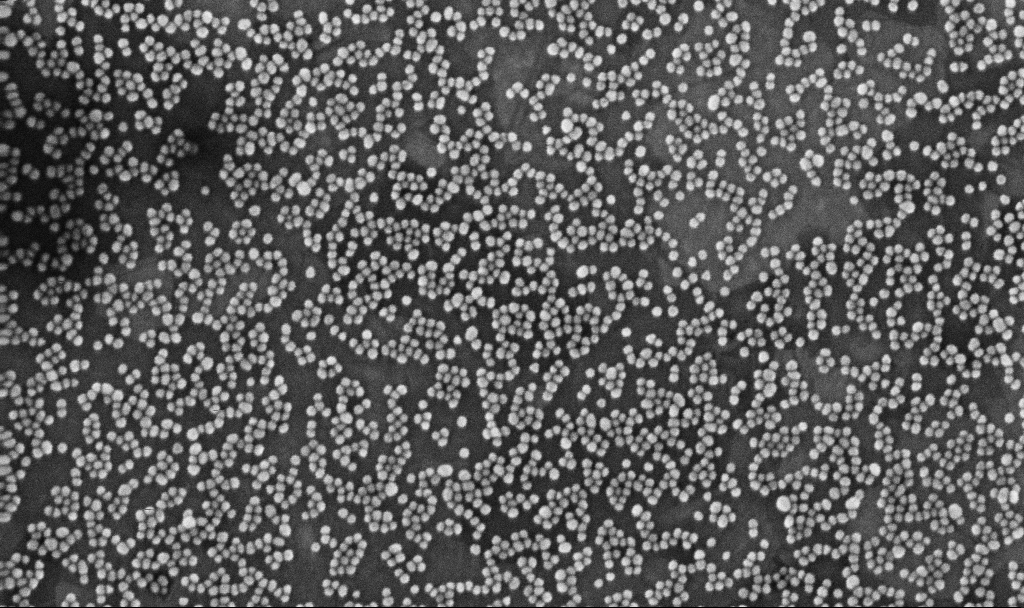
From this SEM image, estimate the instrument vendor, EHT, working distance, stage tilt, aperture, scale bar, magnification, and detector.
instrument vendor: Zeiss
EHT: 10 kV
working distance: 3.3 mm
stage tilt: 30°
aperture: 30 µm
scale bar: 100 nm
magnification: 200 K X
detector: InLens